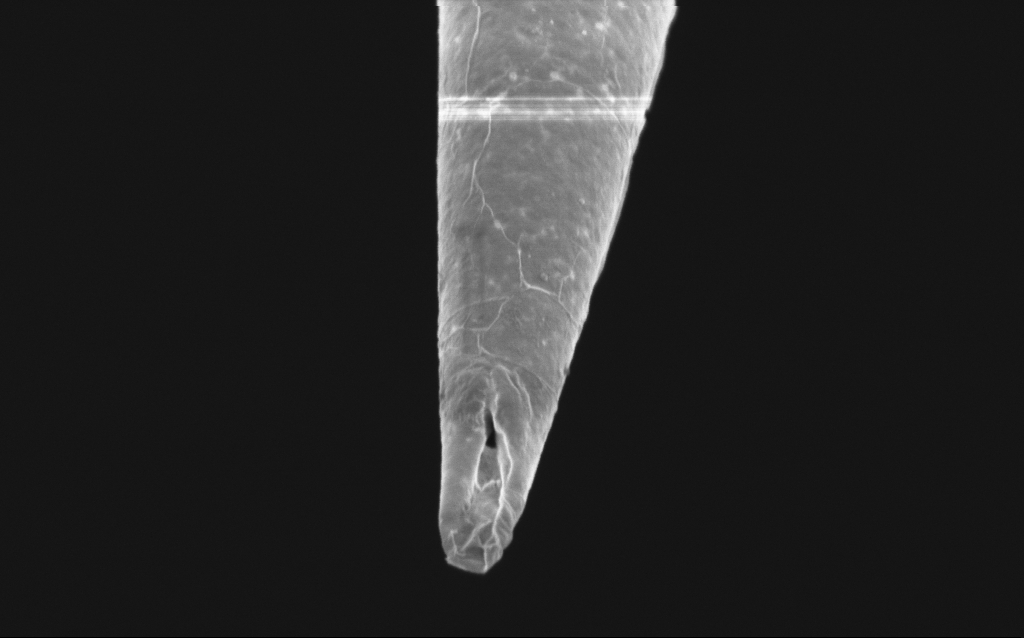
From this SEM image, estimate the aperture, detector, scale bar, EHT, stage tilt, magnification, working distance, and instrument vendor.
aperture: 30 µm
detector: InLens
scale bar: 1000 nm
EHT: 1 kV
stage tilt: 45°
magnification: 42.72 K X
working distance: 6 mm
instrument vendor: Zeiss